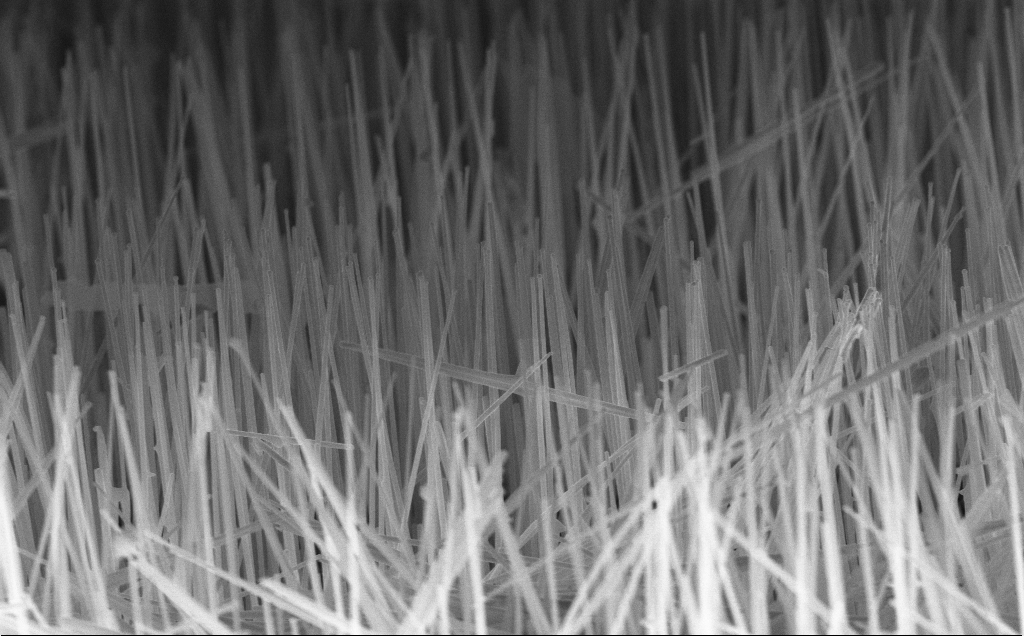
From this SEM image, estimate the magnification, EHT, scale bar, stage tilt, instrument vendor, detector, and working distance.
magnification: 20 K X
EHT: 10 kV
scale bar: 2000 nm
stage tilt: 44.7°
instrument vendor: Zeiss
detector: InLens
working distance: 6 mm